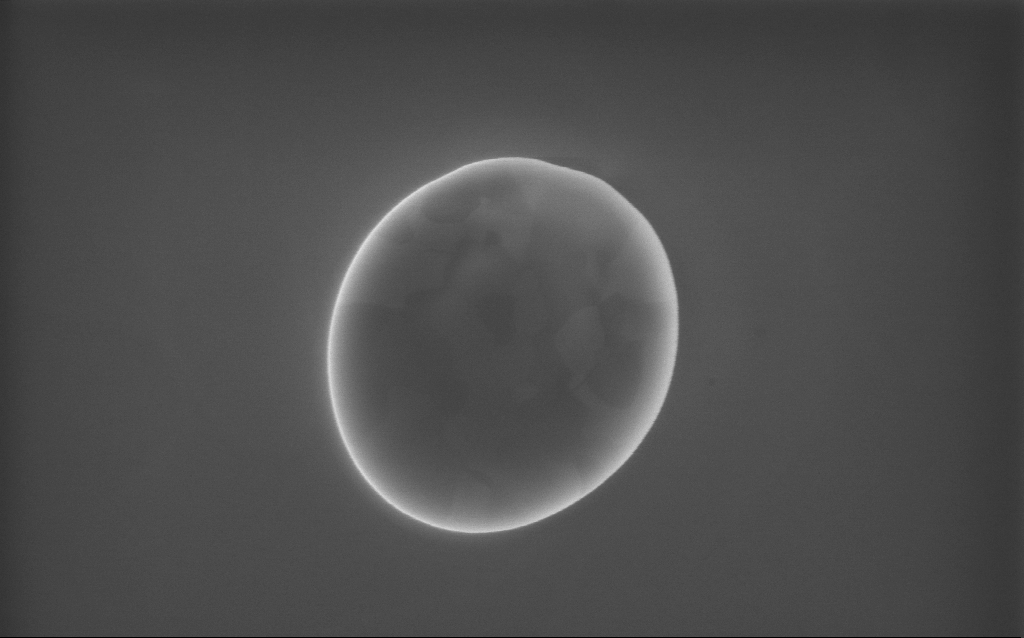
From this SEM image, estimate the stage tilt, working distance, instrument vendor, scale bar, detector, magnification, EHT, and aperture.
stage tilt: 0°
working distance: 3 mm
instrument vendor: Zeiss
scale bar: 1000 nm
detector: InLens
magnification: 70 K X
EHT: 10 kV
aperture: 30 µm